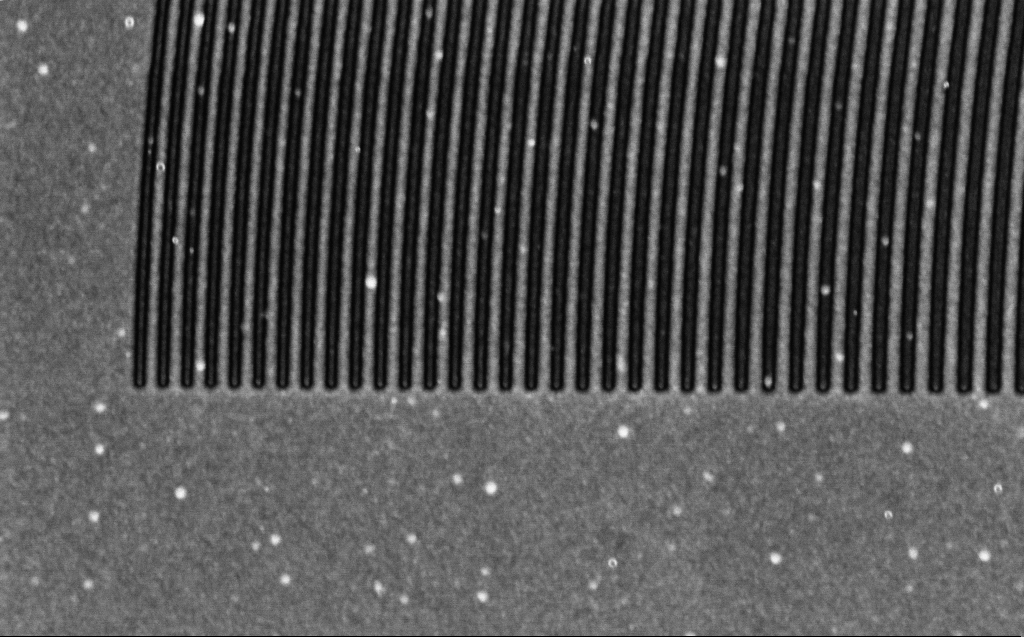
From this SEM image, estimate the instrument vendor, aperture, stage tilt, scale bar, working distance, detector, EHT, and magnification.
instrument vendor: Zeiss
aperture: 30 µm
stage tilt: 30°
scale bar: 2000 nm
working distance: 4 mm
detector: InLens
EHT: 1 kV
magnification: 21.71 K X